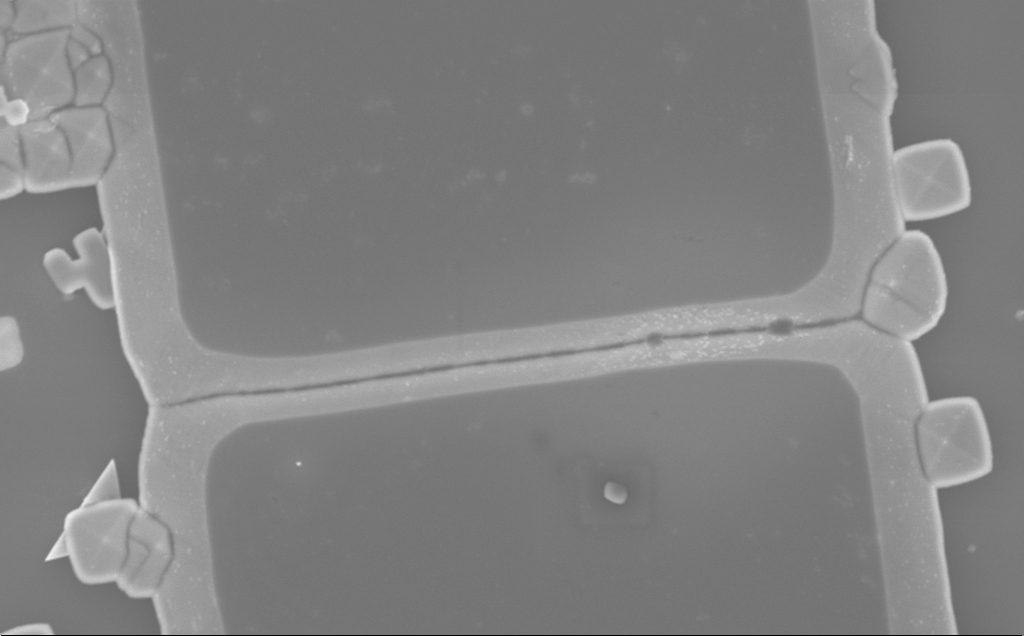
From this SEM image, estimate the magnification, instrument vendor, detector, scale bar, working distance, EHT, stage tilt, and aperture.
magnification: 12.08 K X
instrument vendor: Zeiss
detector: InLens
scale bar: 2000 nm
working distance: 10 mm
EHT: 5 kV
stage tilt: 0°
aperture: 30 µm